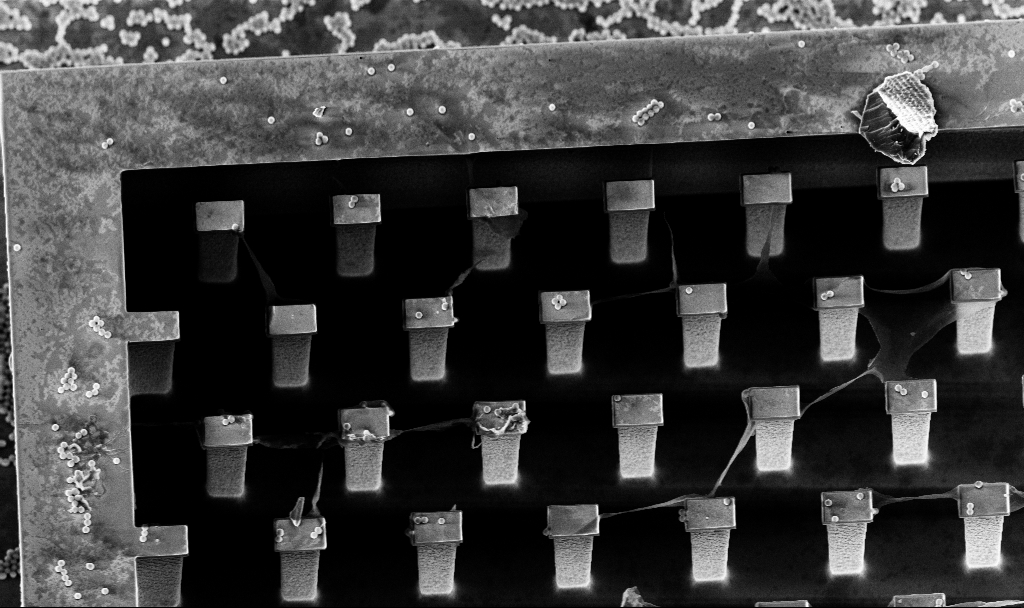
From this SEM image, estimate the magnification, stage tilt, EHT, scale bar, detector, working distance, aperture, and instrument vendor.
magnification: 4.18 K X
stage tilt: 20°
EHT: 5 kV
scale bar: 10000 nm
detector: InLens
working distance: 3.2 mm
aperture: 30 µm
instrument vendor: Zeiss